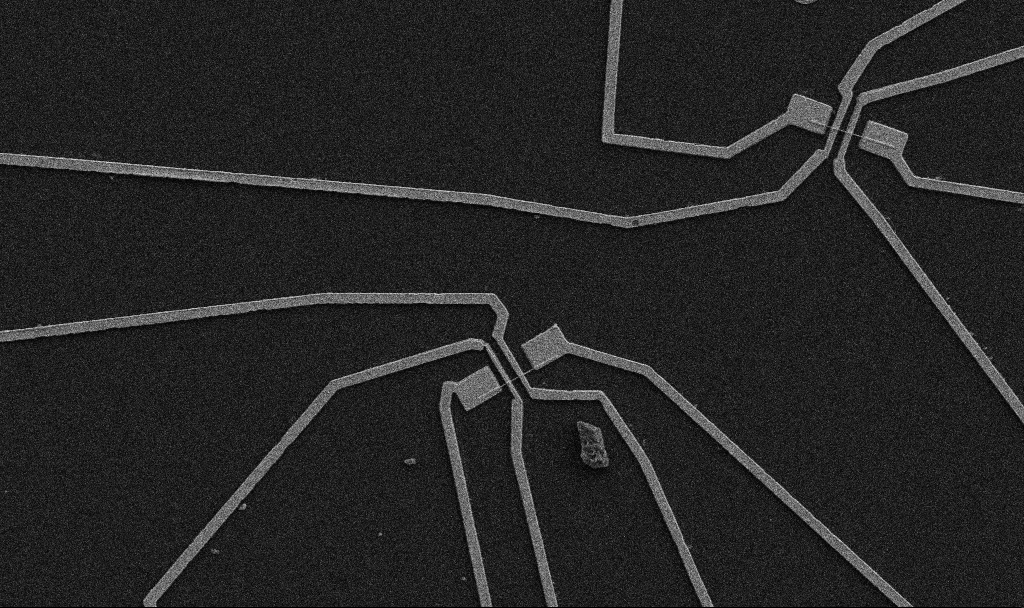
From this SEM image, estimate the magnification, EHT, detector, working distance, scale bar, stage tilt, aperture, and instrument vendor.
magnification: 5 K X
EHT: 5 kV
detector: SE2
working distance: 10.7 mm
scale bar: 10000 nm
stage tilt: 0°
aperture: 30 µm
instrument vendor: Zeiss